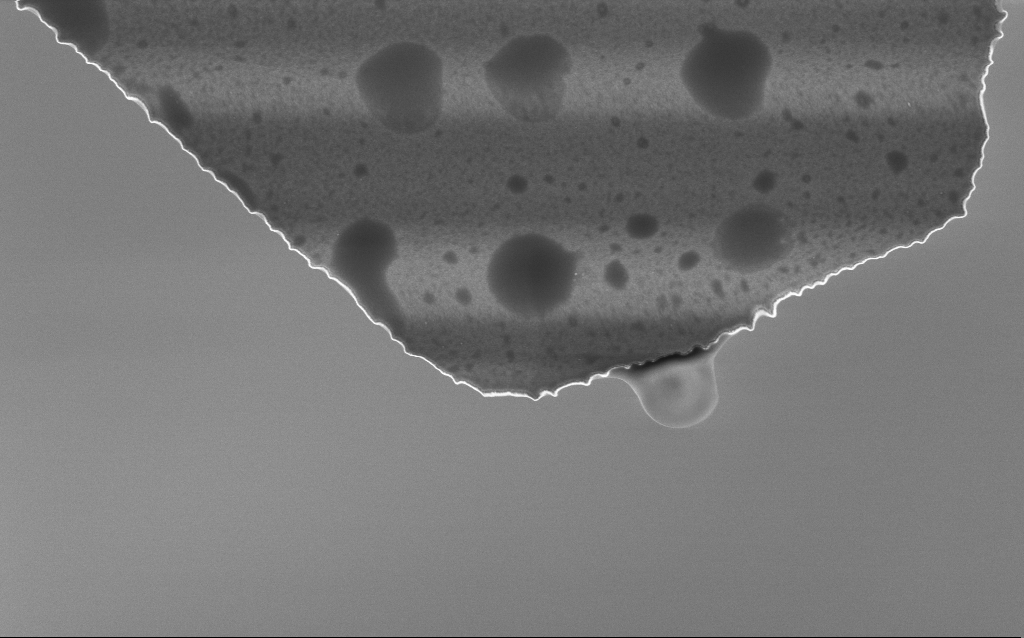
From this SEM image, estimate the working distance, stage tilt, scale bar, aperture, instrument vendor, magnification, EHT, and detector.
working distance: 4 mm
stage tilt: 0.1°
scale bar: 1000 nm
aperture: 30 µm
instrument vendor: Zeiss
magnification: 31.26 K X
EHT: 3 kV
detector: InLens